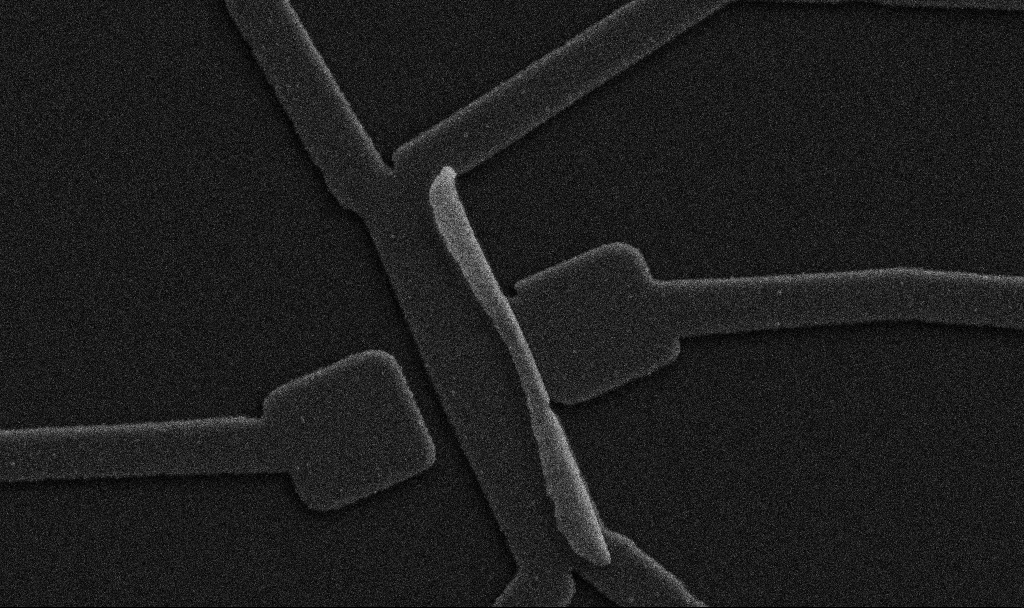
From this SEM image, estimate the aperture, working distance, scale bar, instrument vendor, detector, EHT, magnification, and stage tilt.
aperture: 30 µm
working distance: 10.7 mm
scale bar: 1000 nm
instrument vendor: Zeiss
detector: SE2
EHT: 5 kV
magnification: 20 K X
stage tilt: -0°